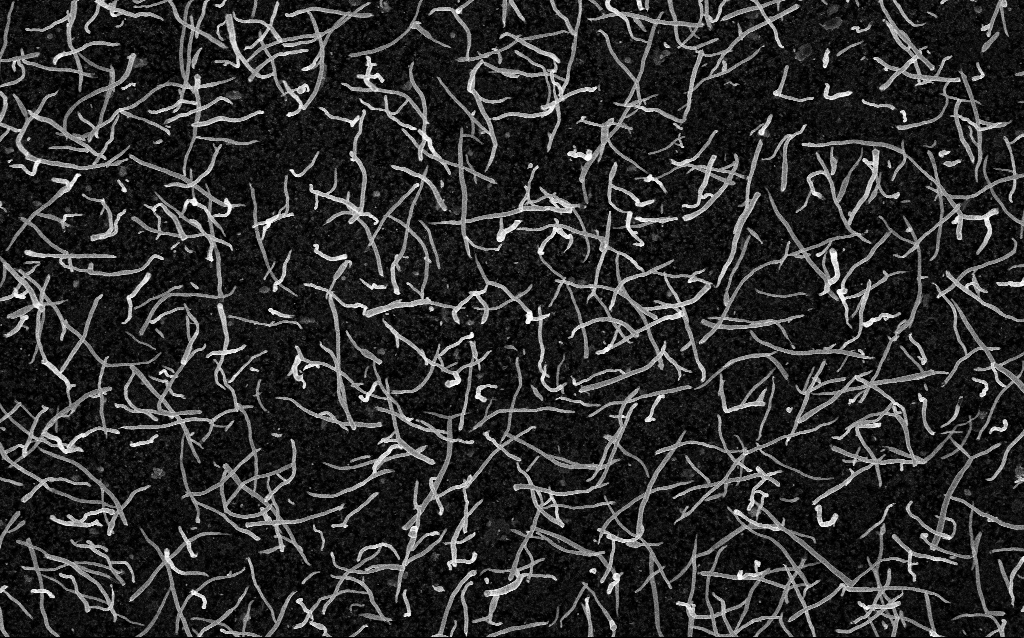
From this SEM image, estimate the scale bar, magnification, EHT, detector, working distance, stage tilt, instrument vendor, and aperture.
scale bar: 1000 nm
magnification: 20 K X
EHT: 5 kV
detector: InLens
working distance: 2.1 mm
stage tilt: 0°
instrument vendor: Zeiss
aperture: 30 µm